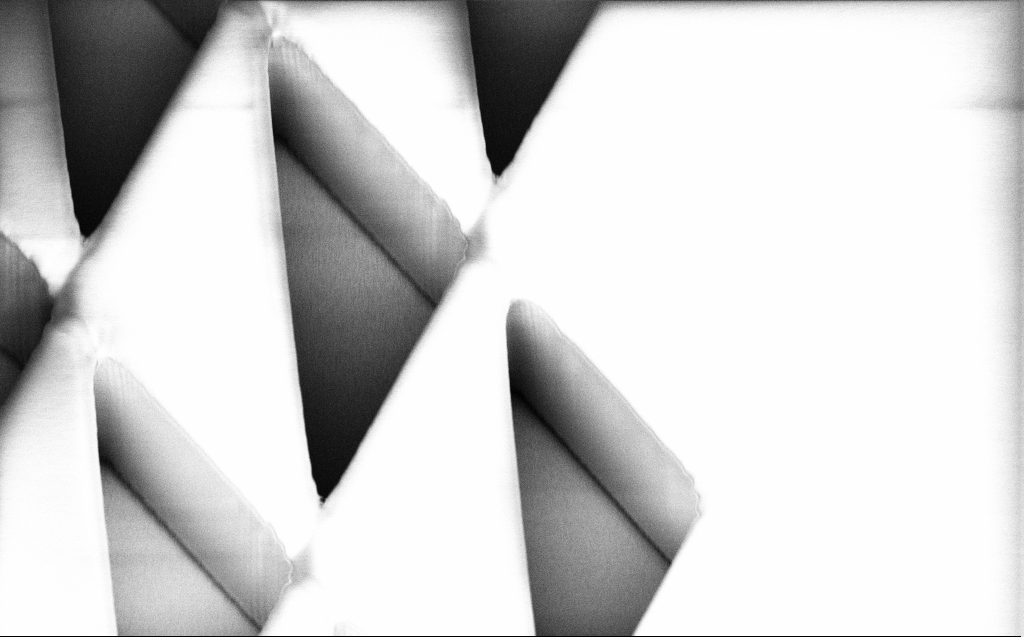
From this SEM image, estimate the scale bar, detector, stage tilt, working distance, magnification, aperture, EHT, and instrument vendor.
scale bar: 20000 nm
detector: InLens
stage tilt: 45°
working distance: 6 mm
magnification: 2.15 K X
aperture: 30 µm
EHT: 1 kV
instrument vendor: Zeiss